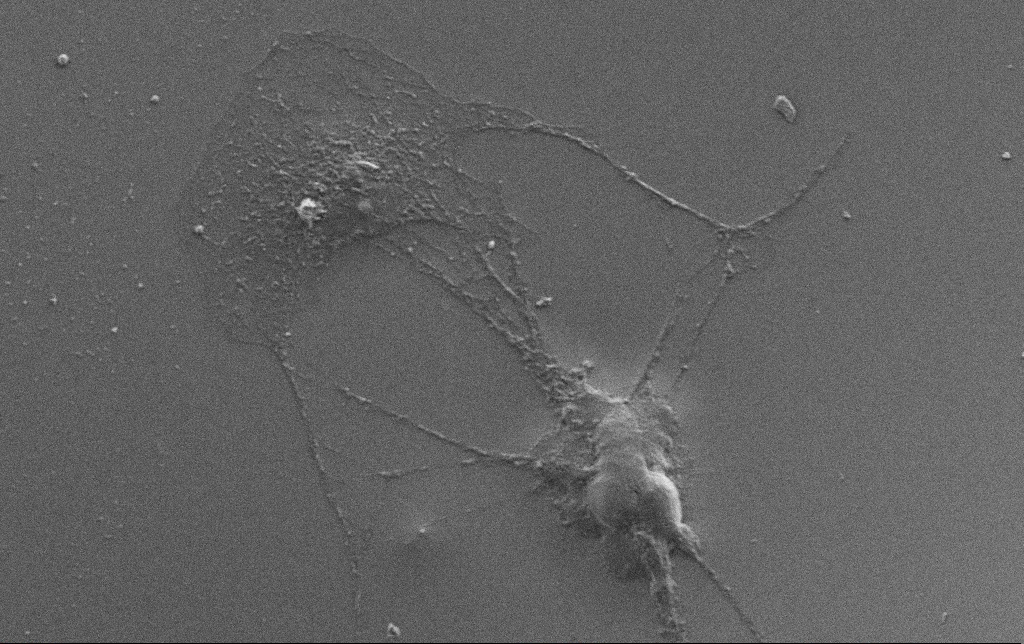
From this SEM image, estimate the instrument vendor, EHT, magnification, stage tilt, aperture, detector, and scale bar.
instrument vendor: Zeiss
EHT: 3 kV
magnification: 2.5 K X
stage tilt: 0°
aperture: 30 µm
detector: SE2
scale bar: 20000 nm